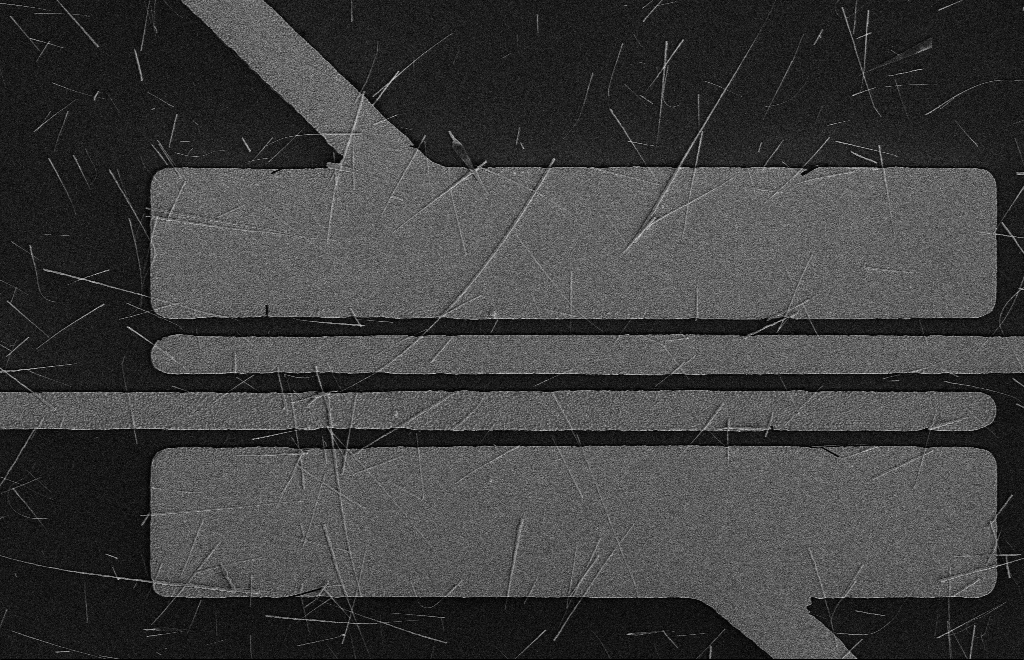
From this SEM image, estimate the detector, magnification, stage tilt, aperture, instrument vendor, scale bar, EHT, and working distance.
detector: SE2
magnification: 5.11 K X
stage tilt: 0°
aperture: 10 µm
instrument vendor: Zeiss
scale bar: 2000 nm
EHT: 5 kV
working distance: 16 mm